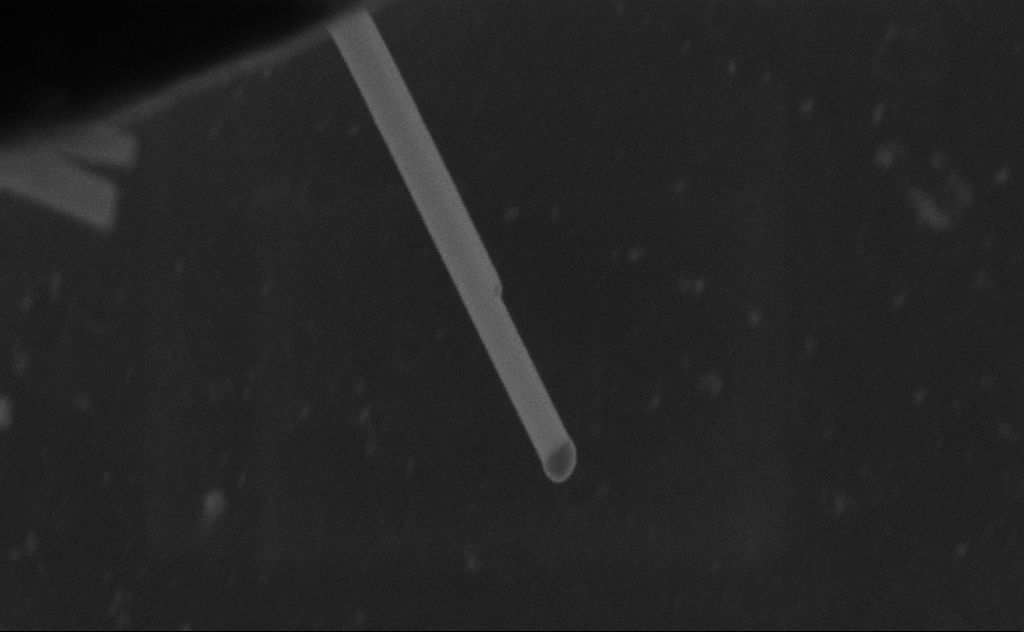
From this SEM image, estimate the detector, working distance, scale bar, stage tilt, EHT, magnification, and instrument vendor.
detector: SE2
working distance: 8 mm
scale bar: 200 nm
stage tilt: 0°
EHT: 20 kV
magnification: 203.24 K X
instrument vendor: Zeiss